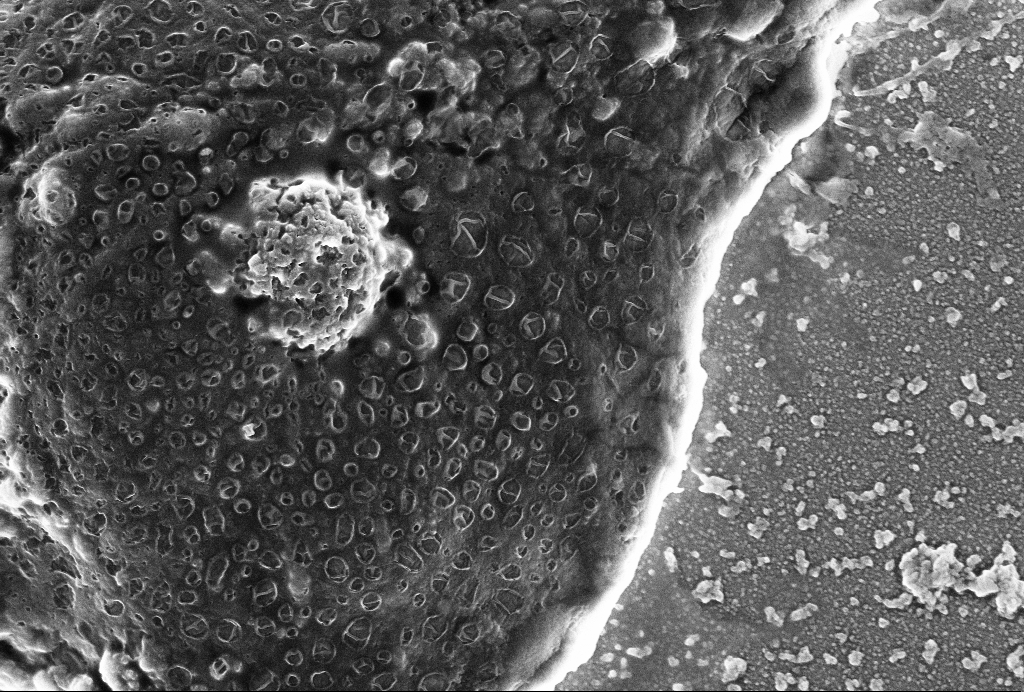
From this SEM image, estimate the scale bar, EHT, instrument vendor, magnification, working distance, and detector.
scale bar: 2000 nm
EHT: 4 kV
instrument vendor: Zeiss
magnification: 25 K X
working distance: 6 mm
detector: SE2